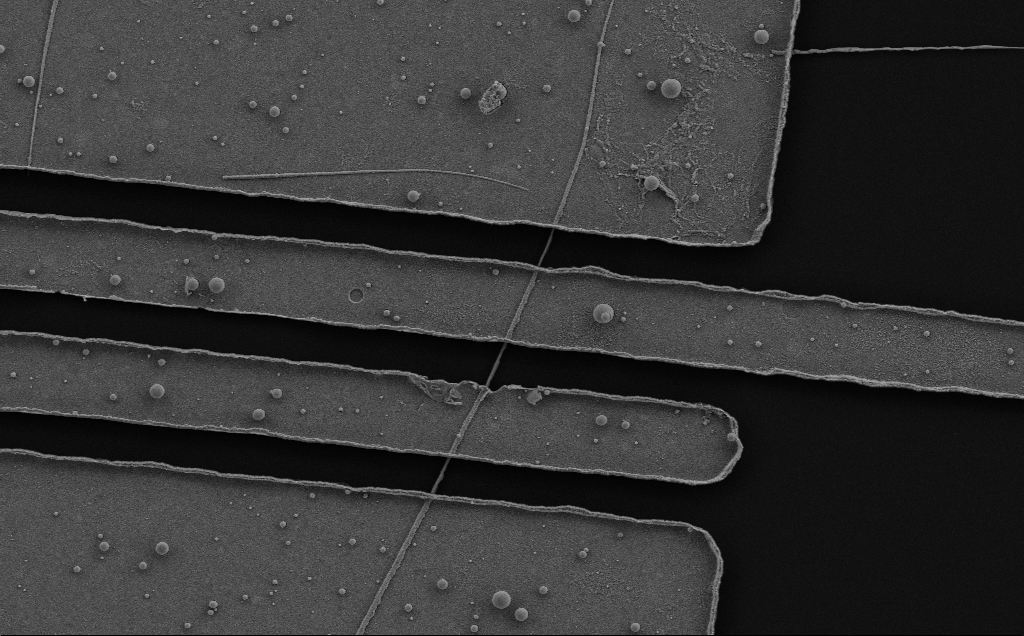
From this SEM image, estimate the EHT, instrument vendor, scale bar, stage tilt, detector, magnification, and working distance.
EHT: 5 kV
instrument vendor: Zeiss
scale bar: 2000 nm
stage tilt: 0°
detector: SE2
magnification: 11.39 K X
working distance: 6 mm